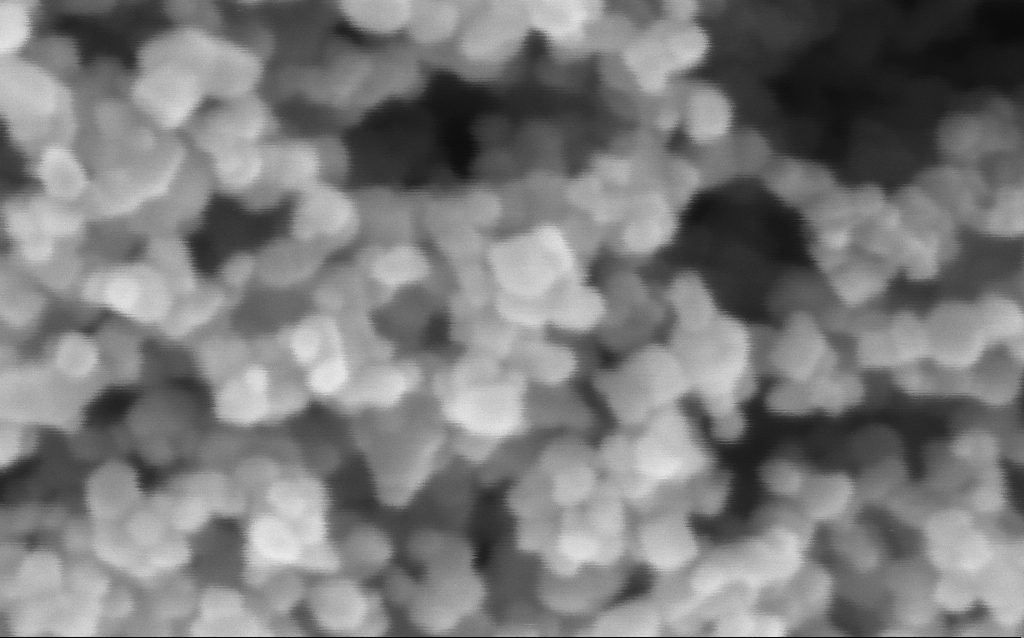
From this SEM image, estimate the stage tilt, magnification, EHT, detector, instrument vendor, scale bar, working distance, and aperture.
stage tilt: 0°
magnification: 716 K X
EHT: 3 kV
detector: InLens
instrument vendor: Zeiss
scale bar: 100 nm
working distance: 7.6 mm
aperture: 30 µm